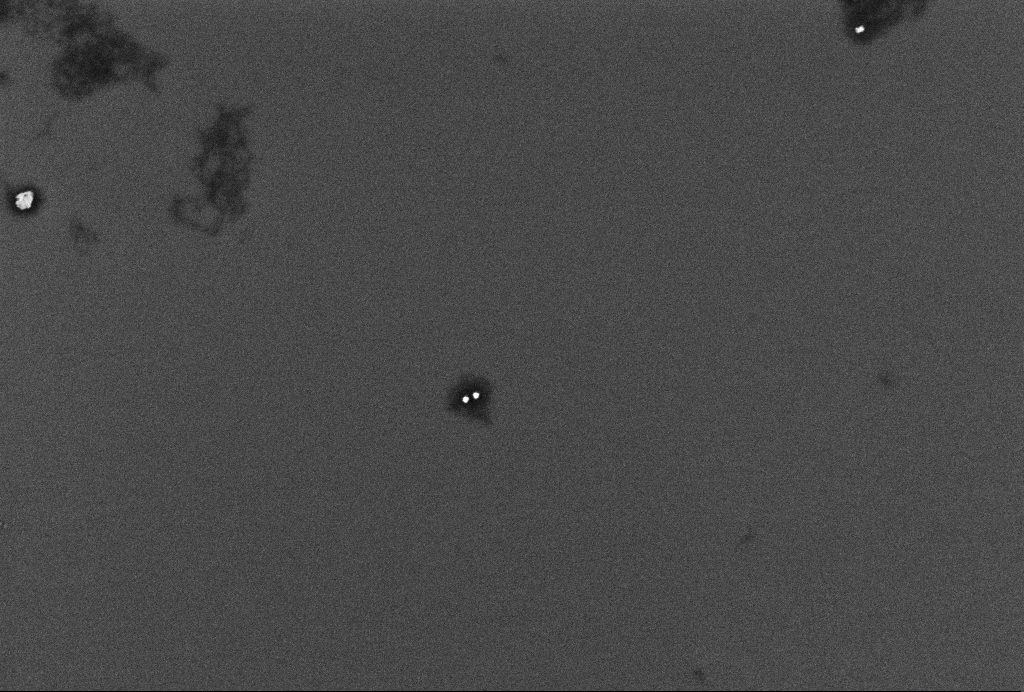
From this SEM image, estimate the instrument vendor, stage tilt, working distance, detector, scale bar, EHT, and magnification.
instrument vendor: Zeiss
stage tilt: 0°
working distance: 3.3 mm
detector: InLens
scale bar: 200 nm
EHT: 2 kV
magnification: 90 K X